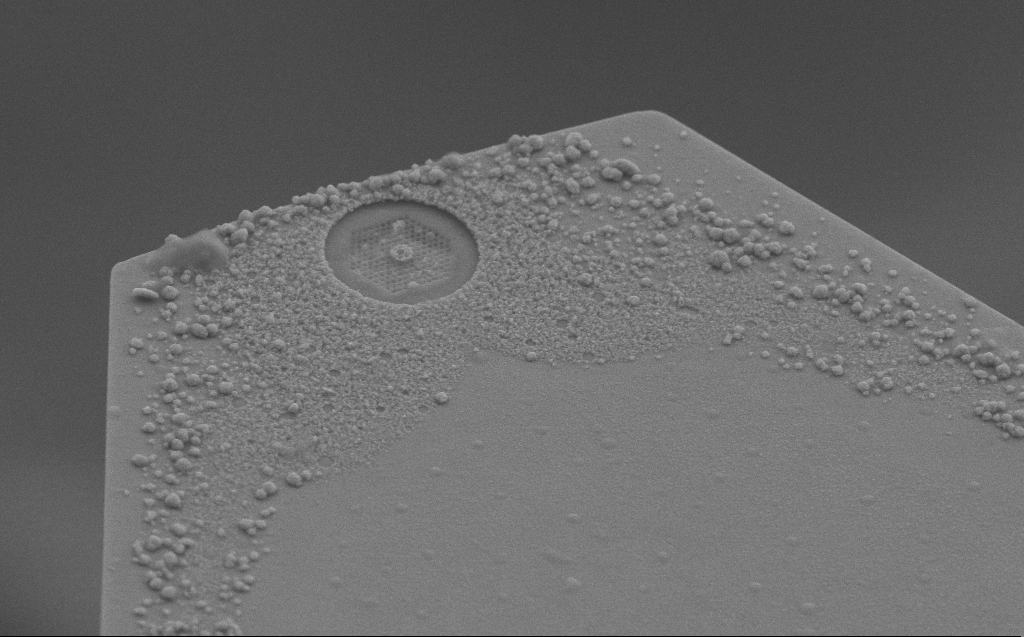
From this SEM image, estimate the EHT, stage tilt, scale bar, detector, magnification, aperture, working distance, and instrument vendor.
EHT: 10 kV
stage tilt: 45°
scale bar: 2000 nm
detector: SE2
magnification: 9.71 K X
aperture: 30 µm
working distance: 6 mm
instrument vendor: Zeiss